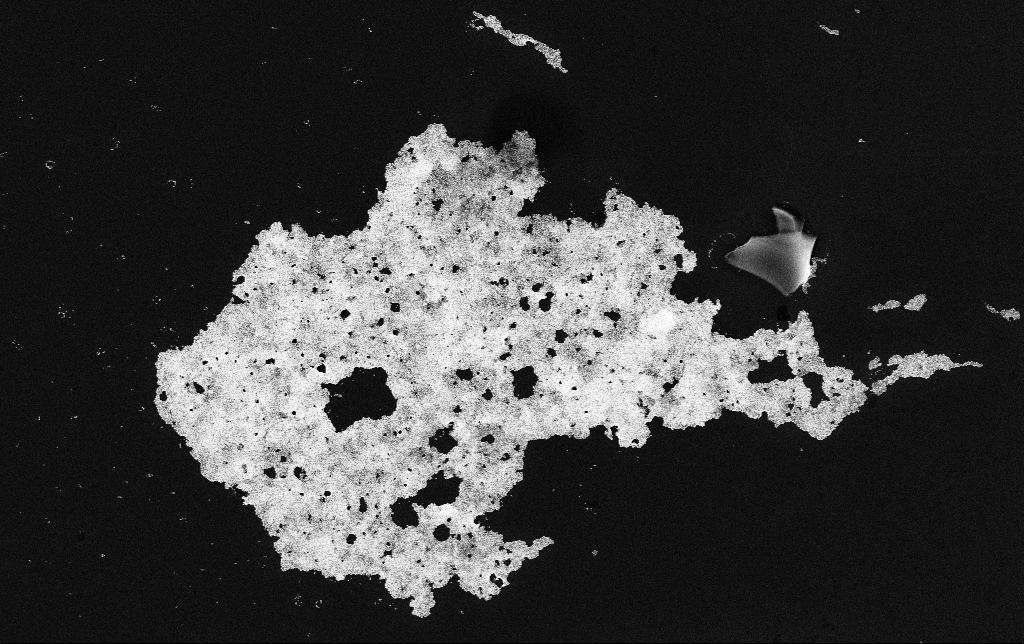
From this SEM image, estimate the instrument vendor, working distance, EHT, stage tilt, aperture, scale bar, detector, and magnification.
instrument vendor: Zeiss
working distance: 3.4 mm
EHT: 10 kV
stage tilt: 0°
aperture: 30 µm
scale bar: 2000 nm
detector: InLens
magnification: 12.5 K X